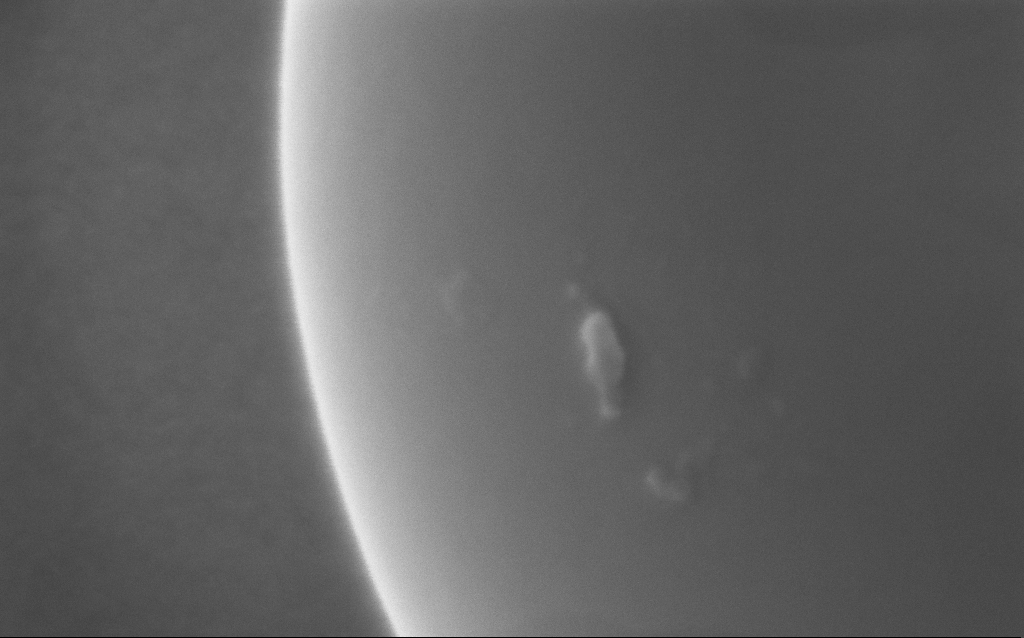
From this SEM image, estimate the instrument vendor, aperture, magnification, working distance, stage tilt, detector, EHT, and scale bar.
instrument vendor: Zeiss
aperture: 30 µm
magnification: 586.85 K X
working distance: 3 mm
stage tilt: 0°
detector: InLens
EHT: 5 kV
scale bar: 100 nm